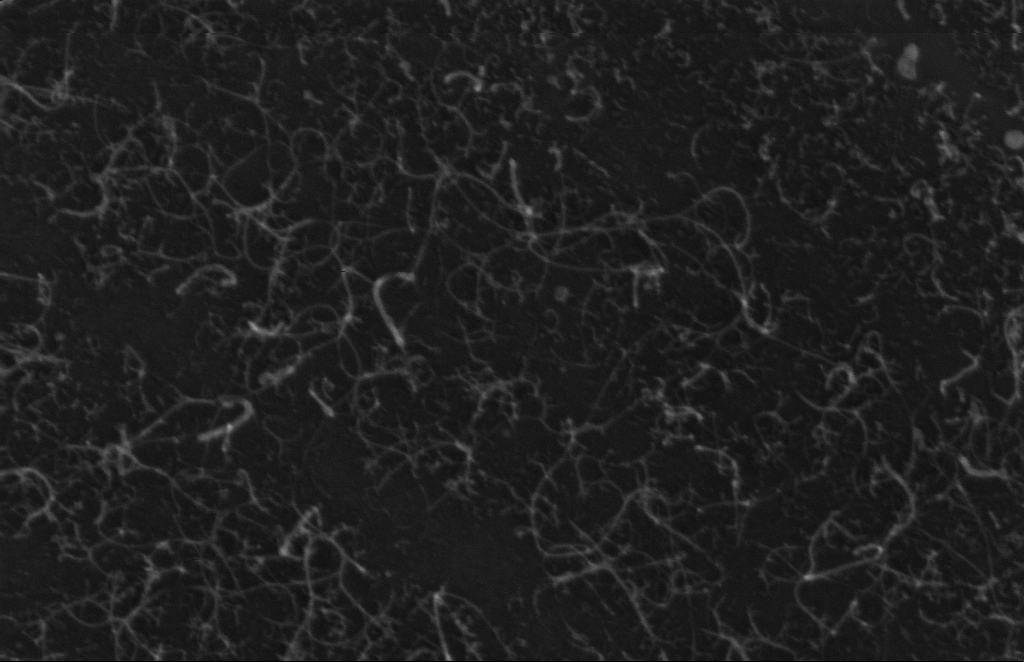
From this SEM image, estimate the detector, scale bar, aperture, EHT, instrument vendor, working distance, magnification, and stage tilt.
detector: InLens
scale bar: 200 nm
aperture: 20 µm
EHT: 5 kV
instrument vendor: Zeiss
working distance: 5 mm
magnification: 228.2 K X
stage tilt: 0°